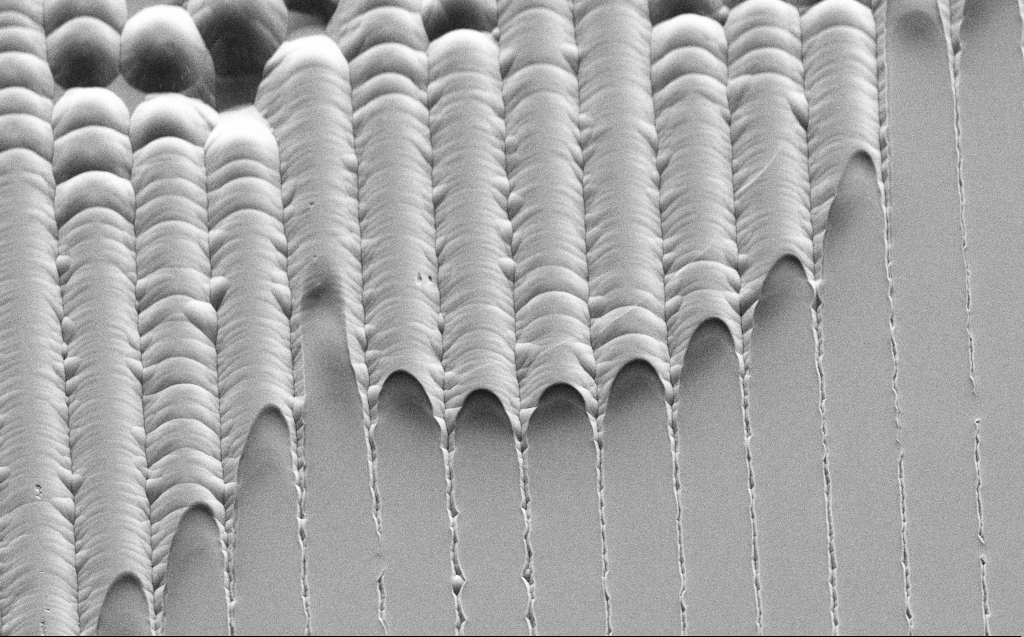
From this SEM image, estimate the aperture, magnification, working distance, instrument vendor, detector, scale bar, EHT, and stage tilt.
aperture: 30 µm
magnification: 2.77 K X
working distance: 9 mm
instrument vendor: Zeiss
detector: SE2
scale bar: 20000 nm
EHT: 3 kV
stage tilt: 45°